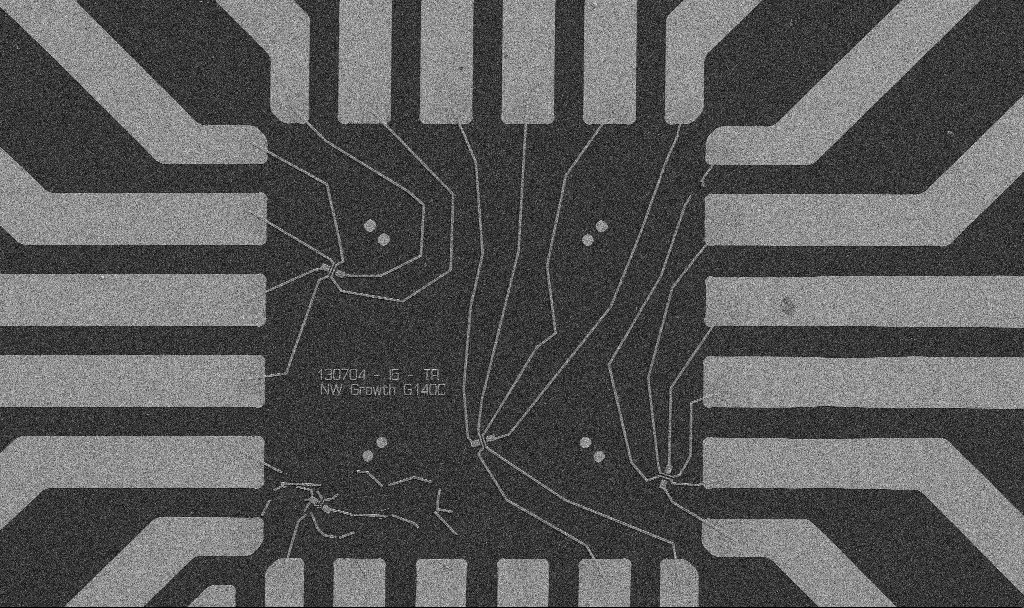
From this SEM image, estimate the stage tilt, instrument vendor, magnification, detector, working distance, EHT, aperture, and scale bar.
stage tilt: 0°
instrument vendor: Zeiss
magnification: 1 K X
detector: SE2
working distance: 8.7 mm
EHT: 5 kV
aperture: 30 µm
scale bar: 20000 nm